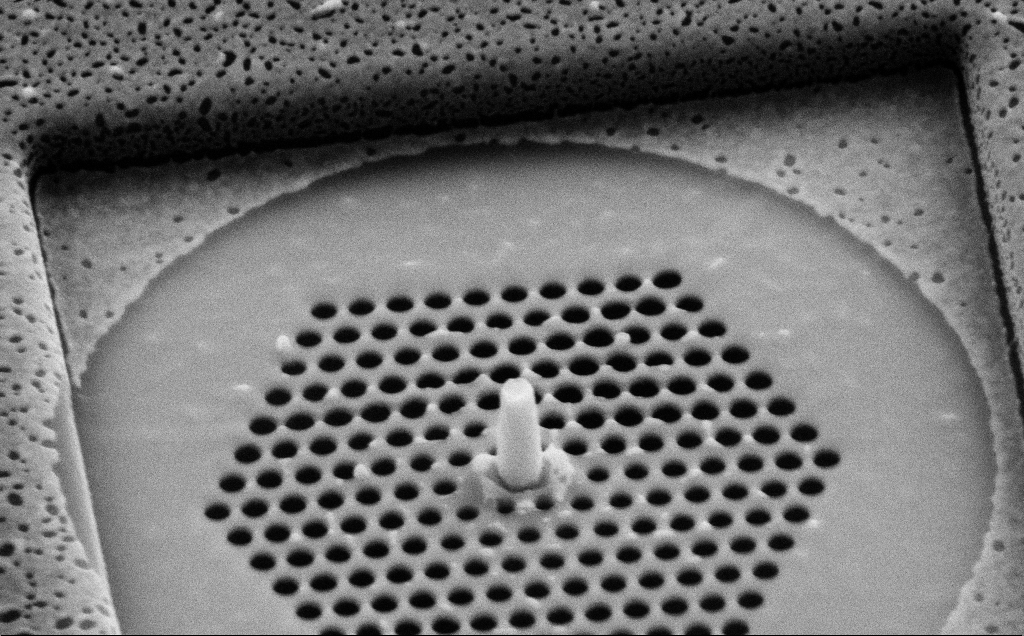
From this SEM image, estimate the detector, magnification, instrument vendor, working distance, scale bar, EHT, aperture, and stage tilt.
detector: SE2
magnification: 56.84 K X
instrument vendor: Zeiss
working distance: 8 mm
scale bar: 1000 nm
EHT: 10 kV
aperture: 30 µm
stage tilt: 45°